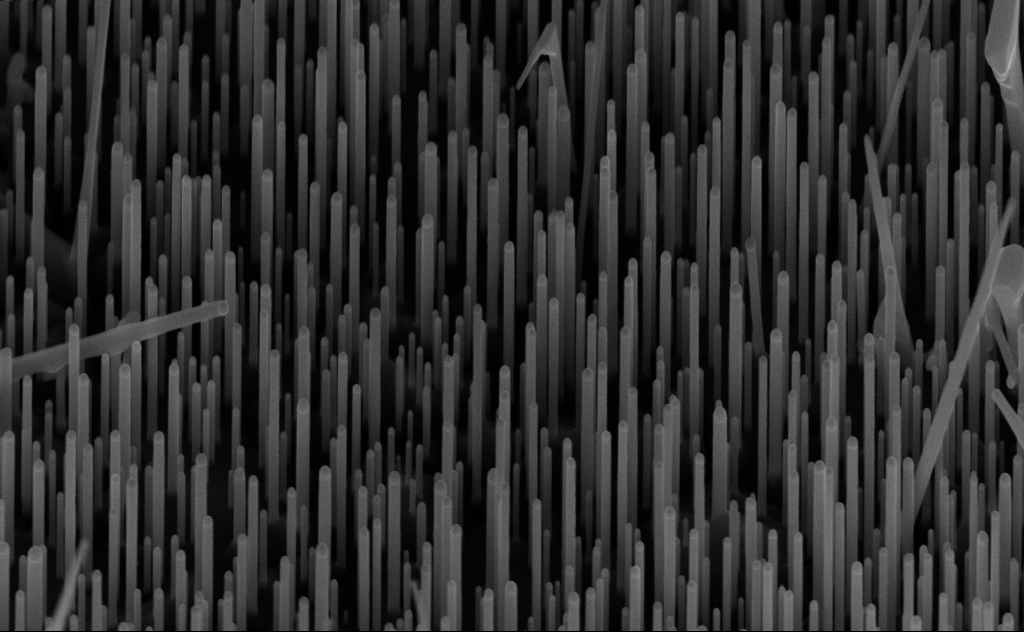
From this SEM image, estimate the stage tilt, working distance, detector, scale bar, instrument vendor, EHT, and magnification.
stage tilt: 45°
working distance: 7 mm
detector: InLens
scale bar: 1000 nm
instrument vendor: Zeiss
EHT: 10 kV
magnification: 62.85 K X